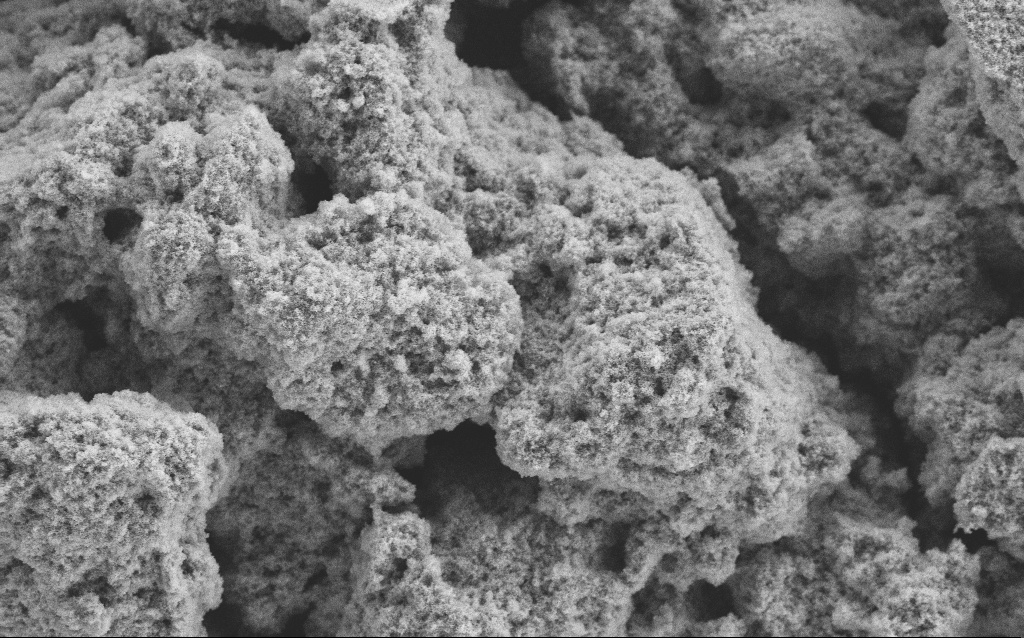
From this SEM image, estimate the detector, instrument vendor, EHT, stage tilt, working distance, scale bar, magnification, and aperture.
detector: SE2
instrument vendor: Zeiss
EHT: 5 kV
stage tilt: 0°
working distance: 4.1 mm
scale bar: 10000 nm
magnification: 6.41 K X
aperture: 30 µm